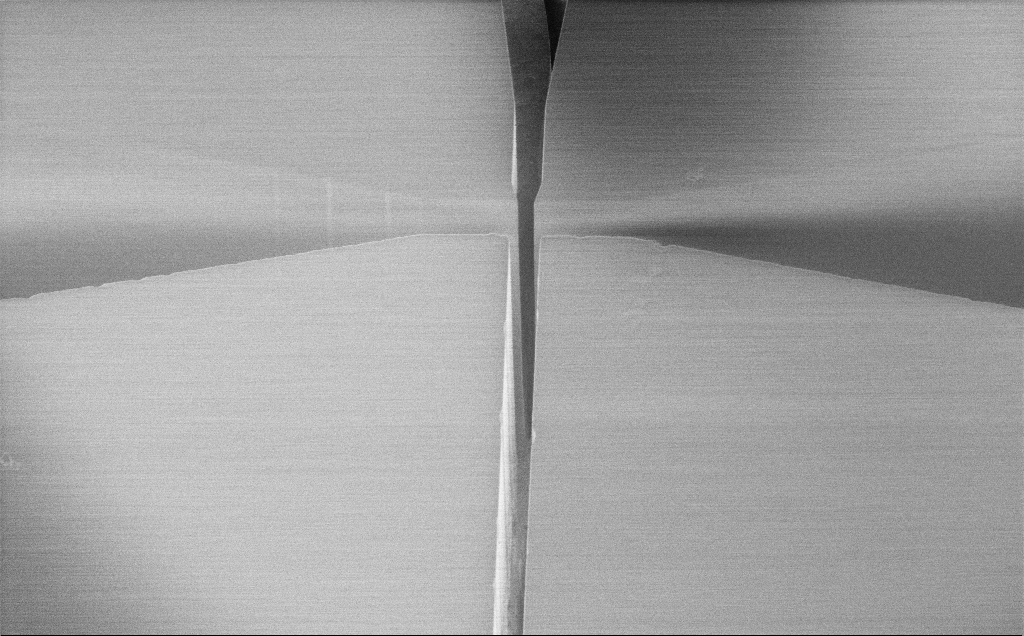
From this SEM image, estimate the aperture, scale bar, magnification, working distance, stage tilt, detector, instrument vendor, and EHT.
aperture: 30 µm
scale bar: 20000 nm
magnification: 0.969 K X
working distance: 6 mm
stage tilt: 45°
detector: InLens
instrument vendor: Zeiss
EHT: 1.2 kV